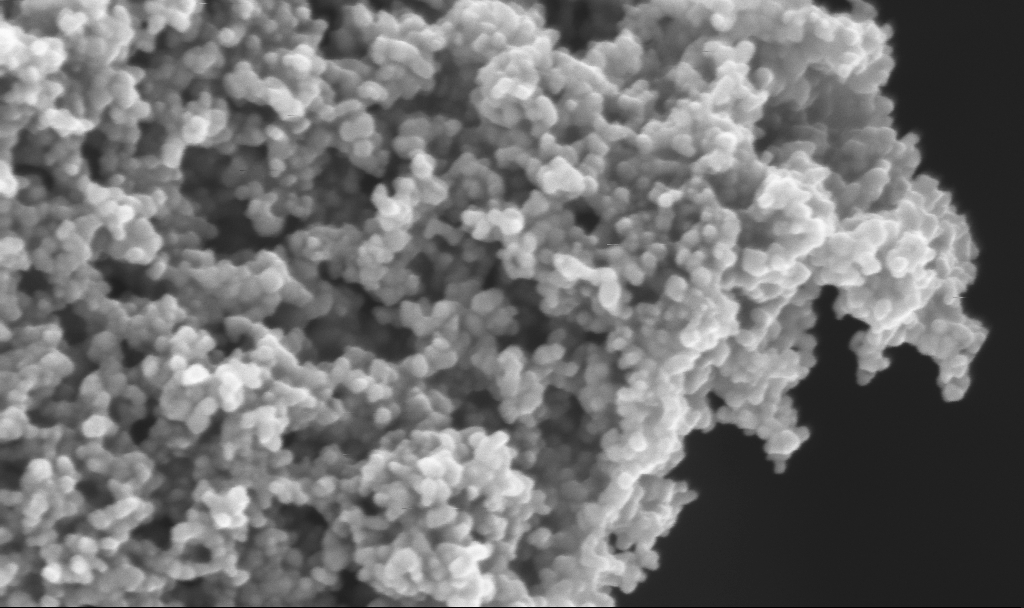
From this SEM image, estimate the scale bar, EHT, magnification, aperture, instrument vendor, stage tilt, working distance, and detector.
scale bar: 200 nm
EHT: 3 kV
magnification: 186.89 K X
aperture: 30 µm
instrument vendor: Zeiss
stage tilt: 0°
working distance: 2.4 mm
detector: InLens